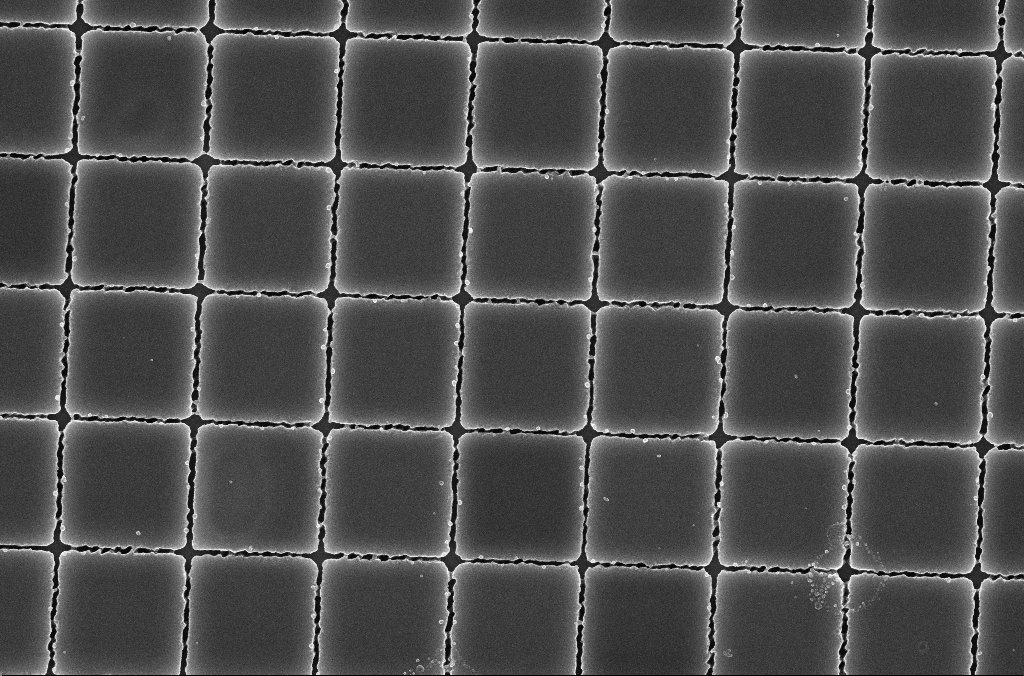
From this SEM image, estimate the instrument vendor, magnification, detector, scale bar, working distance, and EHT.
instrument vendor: Zeiss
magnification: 24.35 K X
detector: InLens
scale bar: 1000 nm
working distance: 4.2 mm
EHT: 5 kV